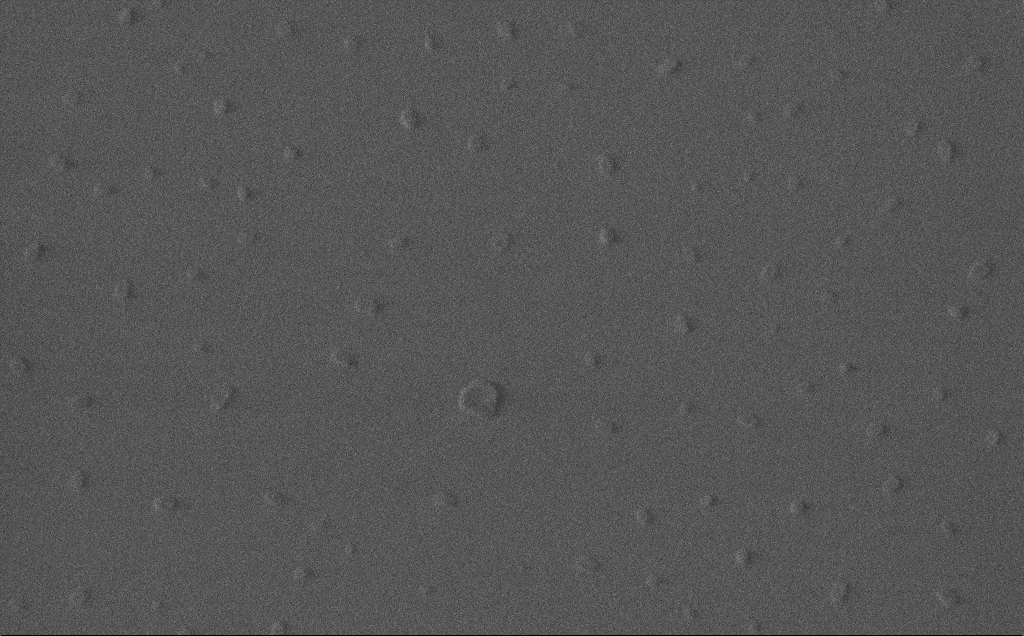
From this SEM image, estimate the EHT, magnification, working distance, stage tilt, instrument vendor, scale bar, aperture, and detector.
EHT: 1 kV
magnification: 79.95 K X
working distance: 3 mm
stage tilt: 0°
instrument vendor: Zeiss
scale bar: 200 nm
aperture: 30 µm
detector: InLens